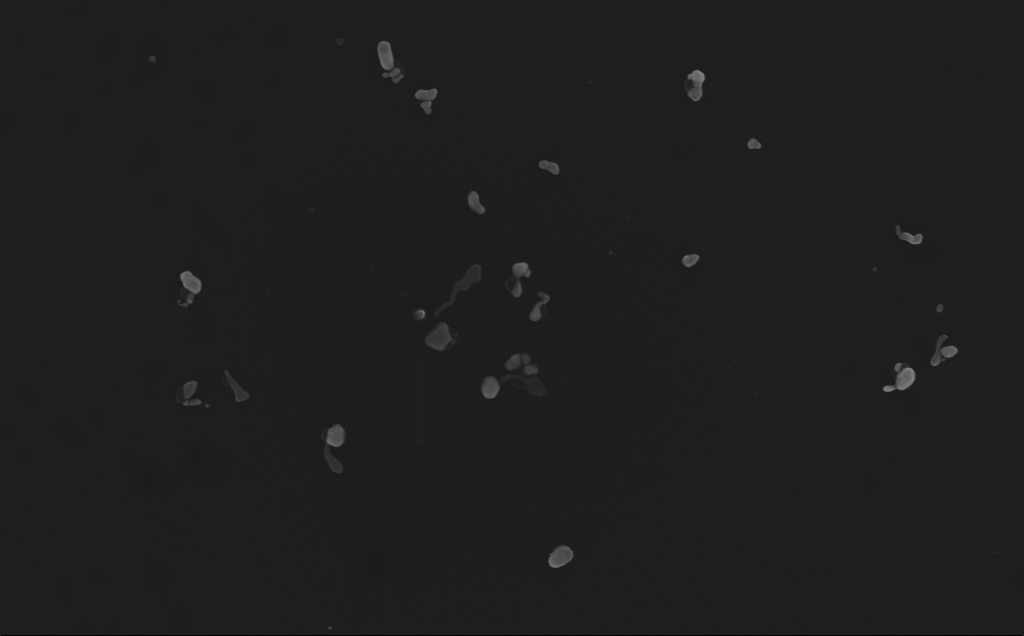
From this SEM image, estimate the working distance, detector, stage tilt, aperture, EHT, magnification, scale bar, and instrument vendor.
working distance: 3 mm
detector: InLens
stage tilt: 0°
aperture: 30 µm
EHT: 10 kV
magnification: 82.75 K X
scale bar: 200 nm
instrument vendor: Zeiss